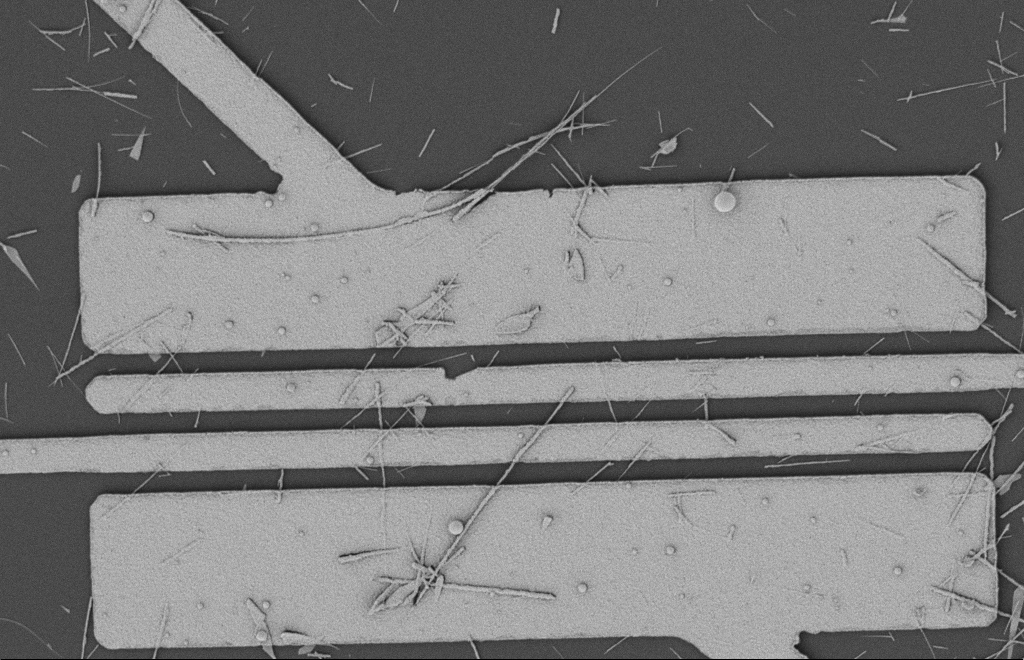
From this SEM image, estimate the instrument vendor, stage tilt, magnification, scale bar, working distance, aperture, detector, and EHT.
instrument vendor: Zeiss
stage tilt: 0°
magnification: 5.4 K X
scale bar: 2000 nm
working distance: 12 mm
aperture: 20 µm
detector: SE2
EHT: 2 kV